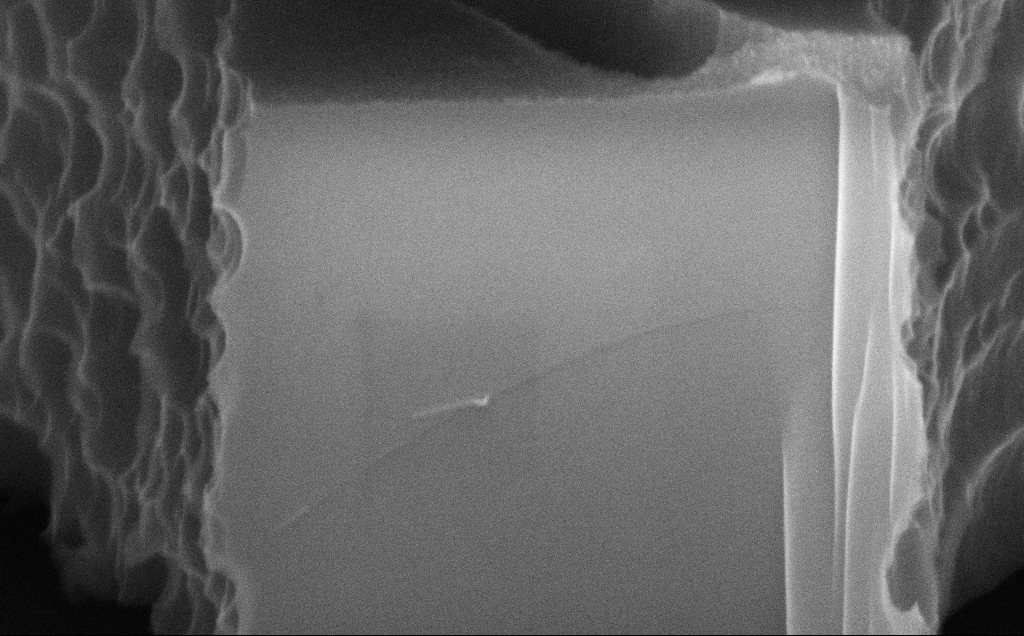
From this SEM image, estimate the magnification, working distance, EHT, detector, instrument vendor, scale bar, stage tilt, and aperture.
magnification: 113.85 K X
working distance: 10 mm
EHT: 10 kV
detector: InLens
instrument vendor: Zeiss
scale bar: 200 nm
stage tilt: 70°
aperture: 30 µm